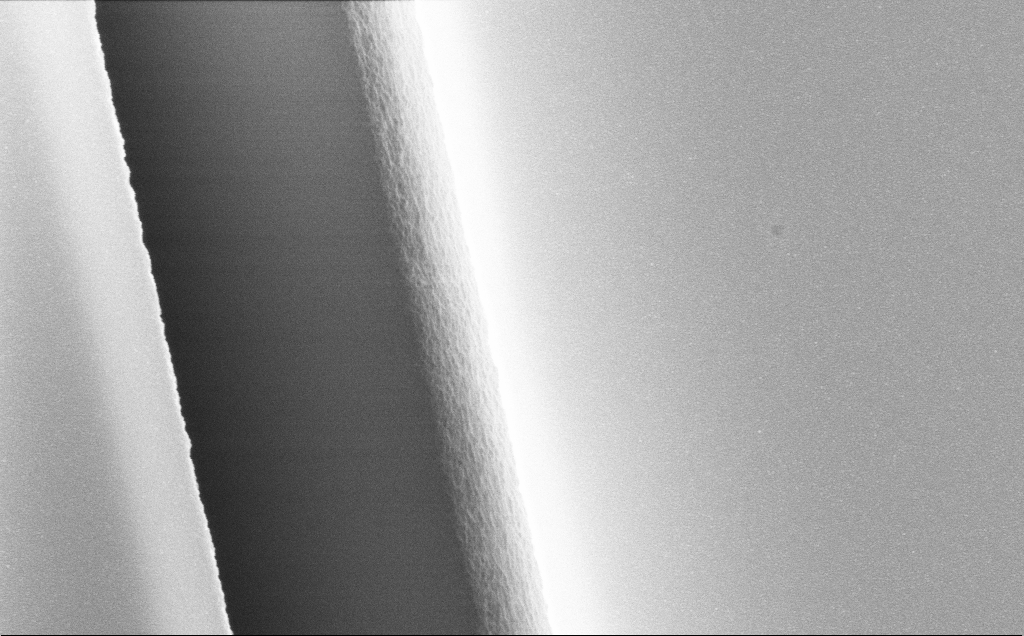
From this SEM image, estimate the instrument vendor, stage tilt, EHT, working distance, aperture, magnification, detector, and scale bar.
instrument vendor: Zeiss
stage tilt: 0°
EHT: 10 kV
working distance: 12 mm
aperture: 30 µm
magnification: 74.23 K X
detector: InLens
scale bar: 200 nm